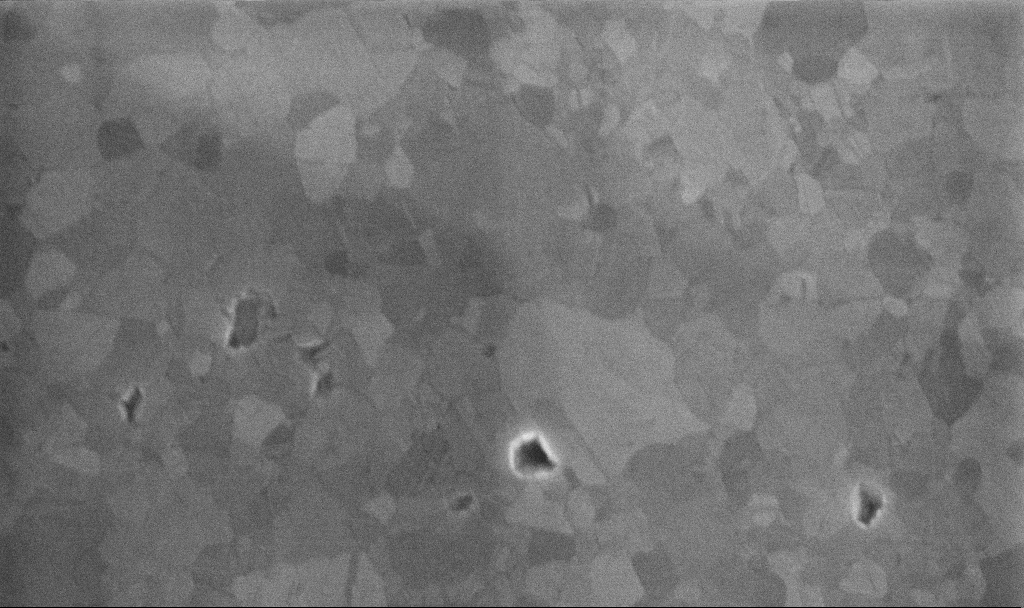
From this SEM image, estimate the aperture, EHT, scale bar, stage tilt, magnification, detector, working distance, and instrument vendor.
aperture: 30 µm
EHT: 10 kV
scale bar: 200 nm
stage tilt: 0°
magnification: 105.49 K X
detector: InLens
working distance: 3.3 mm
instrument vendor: Zeiss